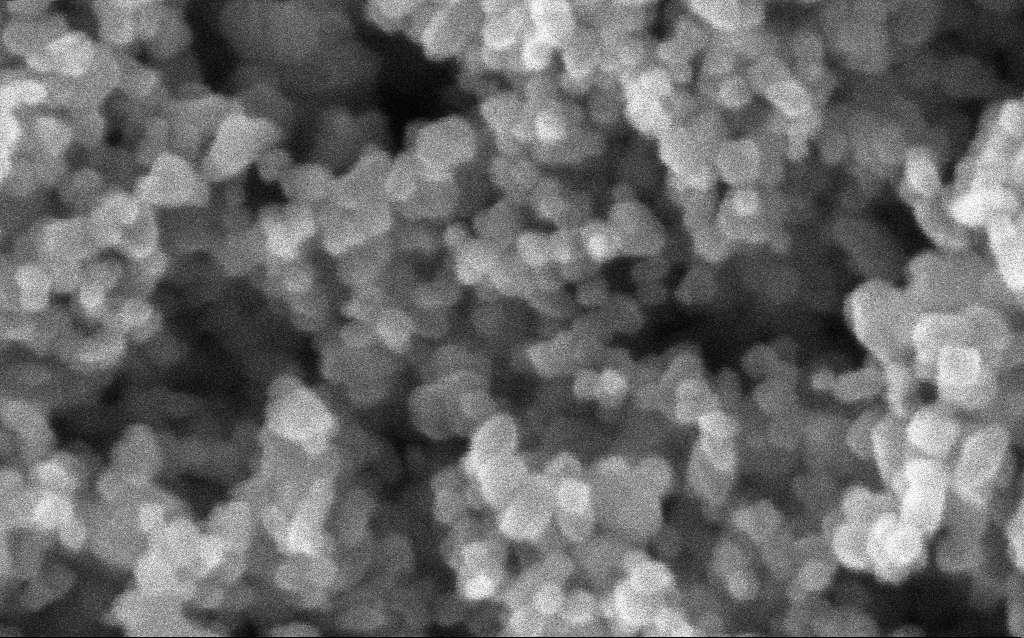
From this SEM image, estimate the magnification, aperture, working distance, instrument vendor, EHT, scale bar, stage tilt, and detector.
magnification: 600 K X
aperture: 30 µm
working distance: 2.9 mm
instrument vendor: Zeiss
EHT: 5 kV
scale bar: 100 nm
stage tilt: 0°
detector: InLens